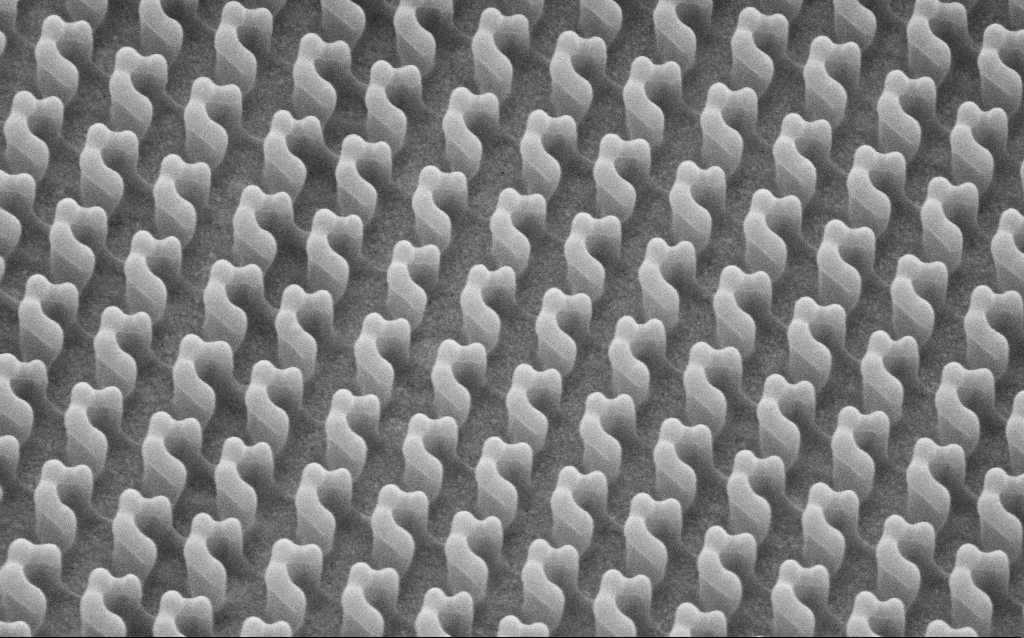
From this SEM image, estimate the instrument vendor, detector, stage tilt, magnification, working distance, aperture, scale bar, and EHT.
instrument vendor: Zeiss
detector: SE2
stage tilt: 30°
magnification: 64.18 K X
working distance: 7.6 mm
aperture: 30 µm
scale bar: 1000 nm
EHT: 5 kV